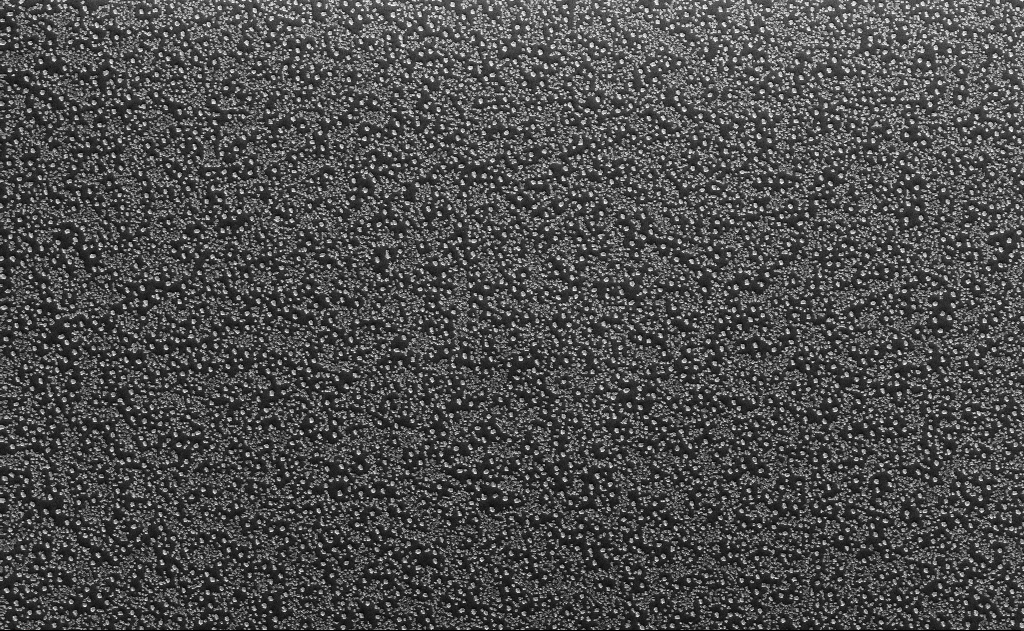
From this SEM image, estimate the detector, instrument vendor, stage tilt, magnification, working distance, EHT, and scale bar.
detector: InLens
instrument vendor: Zeiss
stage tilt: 0°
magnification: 3.36 K X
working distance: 18 mm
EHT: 10 kV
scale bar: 20000 nm